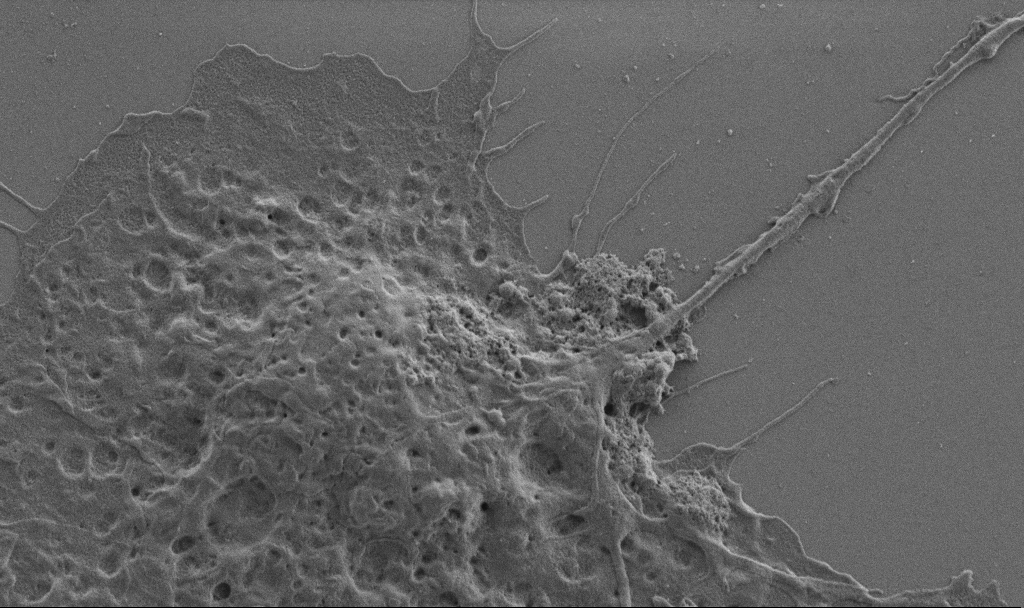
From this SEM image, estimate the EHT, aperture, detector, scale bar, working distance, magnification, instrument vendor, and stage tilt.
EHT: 1 kV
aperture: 30 µm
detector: SE2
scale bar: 2000 nm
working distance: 6.9 mm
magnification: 10 K X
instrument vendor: Zeiss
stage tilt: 0°